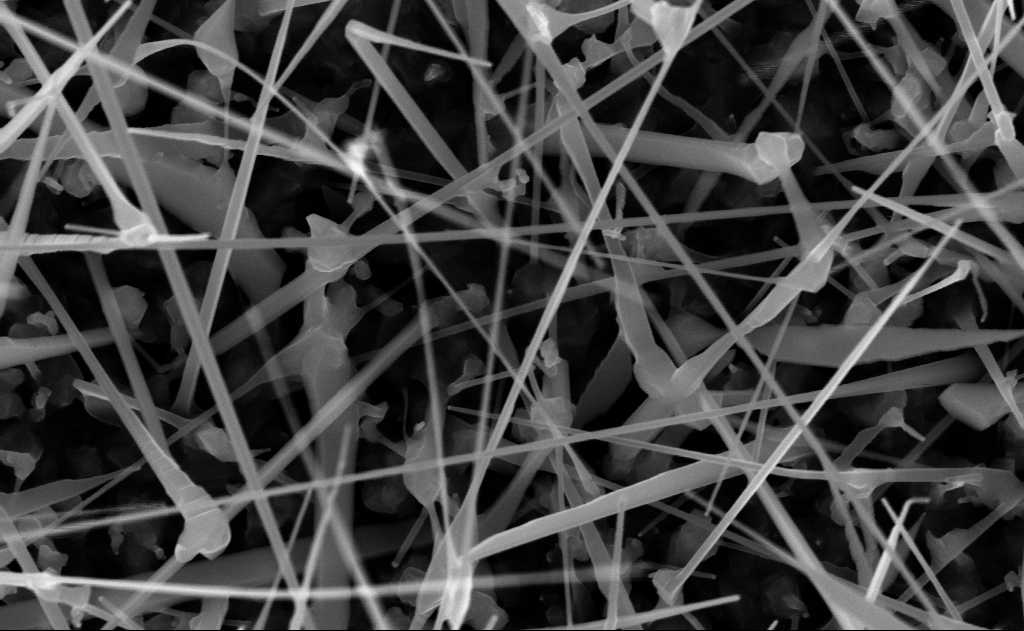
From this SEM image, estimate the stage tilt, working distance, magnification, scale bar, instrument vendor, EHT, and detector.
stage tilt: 0°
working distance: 10 mm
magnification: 80 K X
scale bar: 200 nm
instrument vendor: Zeiss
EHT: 10 kV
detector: InLens